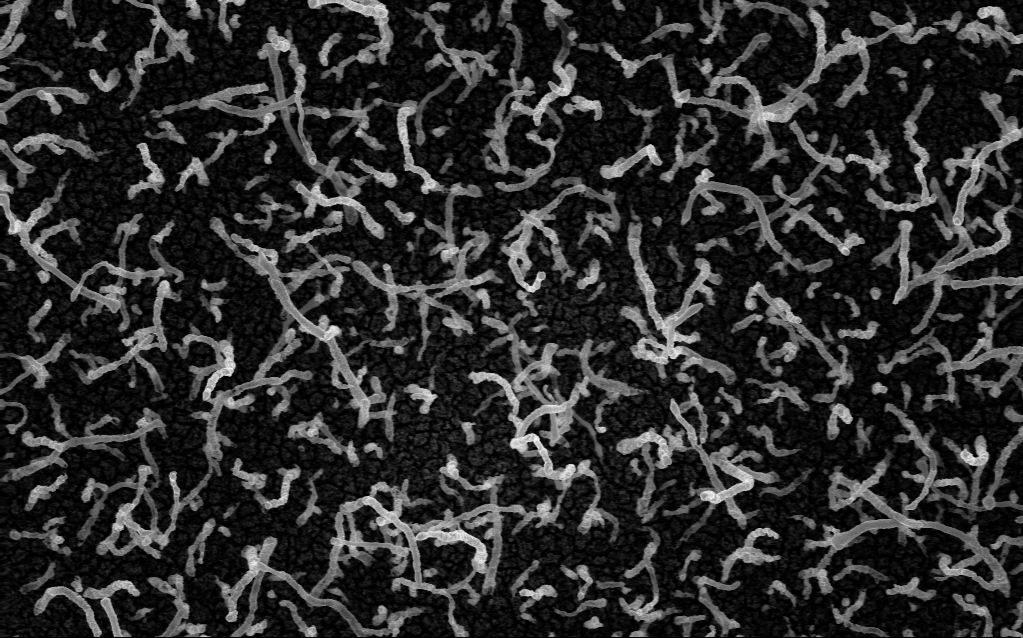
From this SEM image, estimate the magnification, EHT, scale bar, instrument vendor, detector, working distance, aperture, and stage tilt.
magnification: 50 K X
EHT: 5 kV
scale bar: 1000 nm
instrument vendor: Zeiss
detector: InLens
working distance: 2.1 mm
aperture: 30 µm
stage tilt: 0°